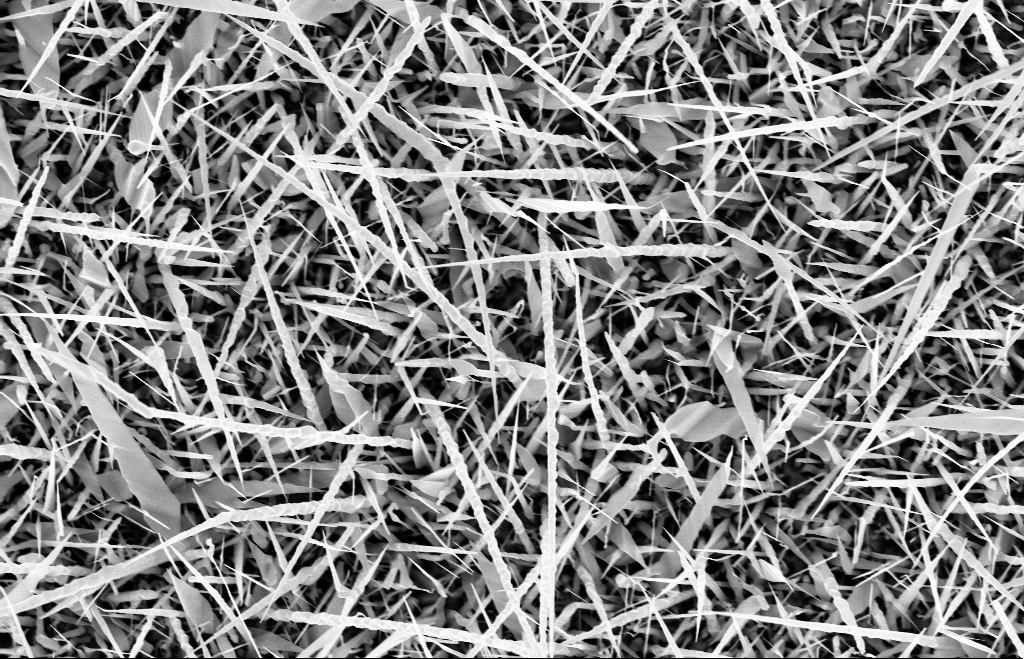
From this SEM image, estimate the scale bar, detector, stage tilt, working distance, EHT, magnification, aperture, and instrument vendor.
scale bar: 1000 nm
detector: InLens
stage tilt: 0°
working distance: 9 mm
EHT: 10 kV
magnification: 20 K X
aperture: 30 µm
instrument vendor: Zeiss